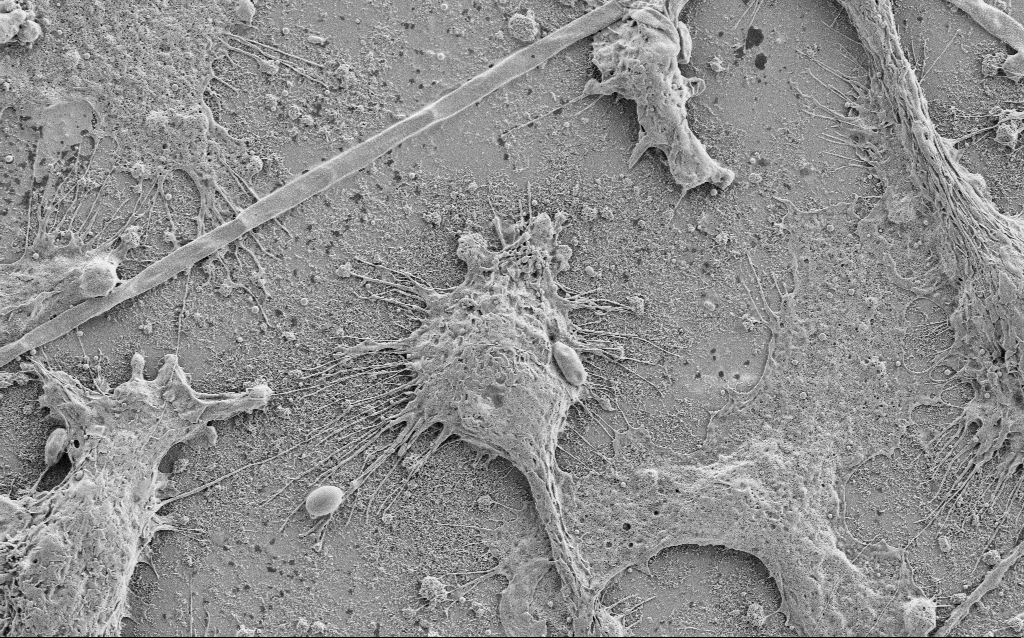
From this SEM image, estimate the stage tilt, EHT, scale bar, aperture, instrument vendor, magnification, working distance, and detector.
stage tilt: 0°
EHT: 2 kV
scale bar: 10000 nm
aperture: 30 µm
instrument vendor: Zeiss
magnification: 3.5 K X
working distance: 6.8 mm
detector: SE2